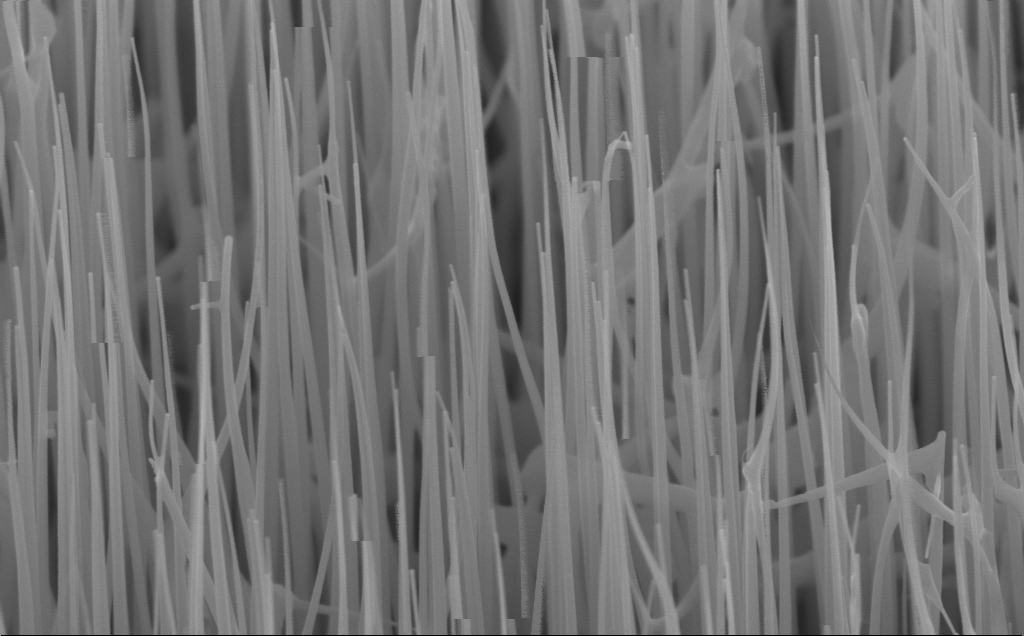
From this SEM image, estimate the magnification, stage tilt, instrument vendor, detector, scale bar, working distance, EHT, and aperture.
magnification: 89.81 K X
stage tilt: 45°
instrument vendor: Zeiss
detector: InLens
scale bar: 200 nm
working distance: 6 mm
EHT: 10 kV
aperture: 30 µm